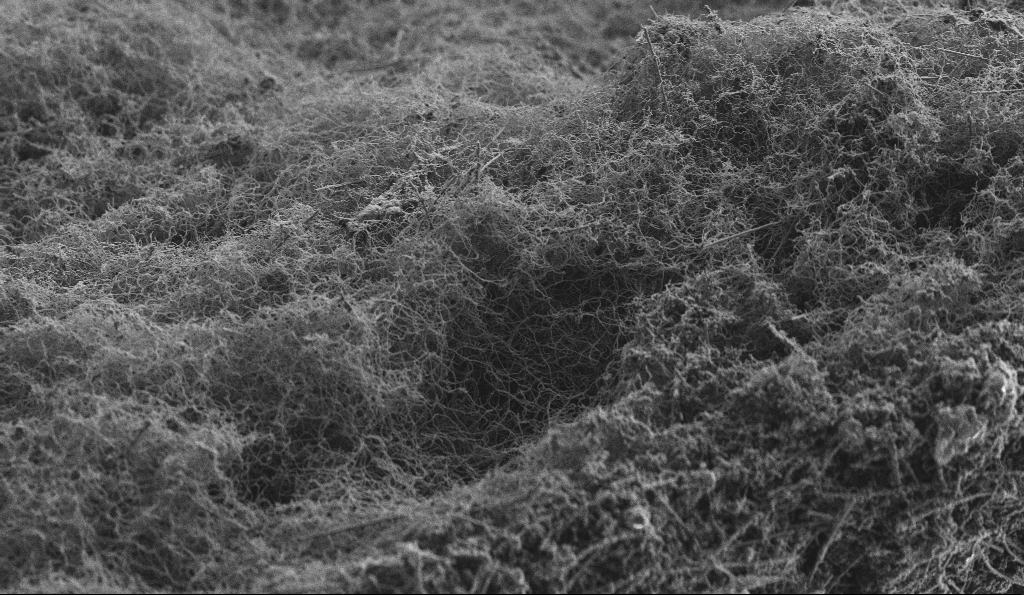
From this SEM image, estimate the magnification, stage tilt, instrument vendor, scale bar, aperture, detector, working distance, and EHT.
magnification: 2 K X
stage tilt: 0°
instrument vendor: Zeiss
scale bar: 10000 nm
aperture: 30 µm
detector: SE2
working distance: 4.6 mm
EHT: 3 kV